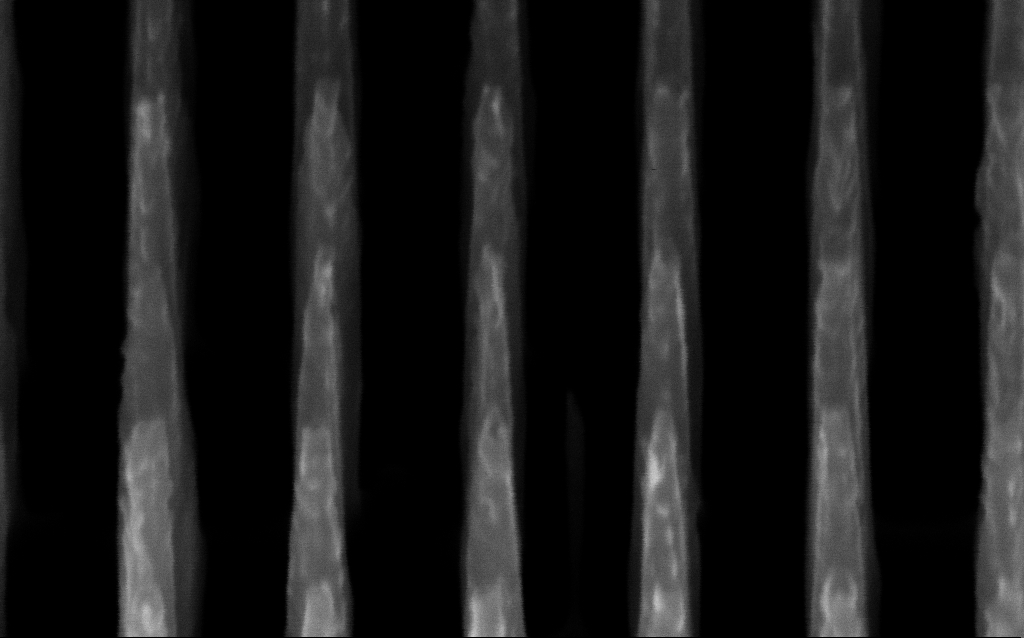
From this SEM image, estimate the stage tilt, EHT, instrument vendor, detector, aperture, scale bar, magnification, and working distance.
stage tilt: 45°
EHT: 3 kV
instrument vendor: Zeiss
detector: InLens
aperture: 30 µm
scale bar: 200 nm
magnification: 319.23 K X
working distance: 5 mm